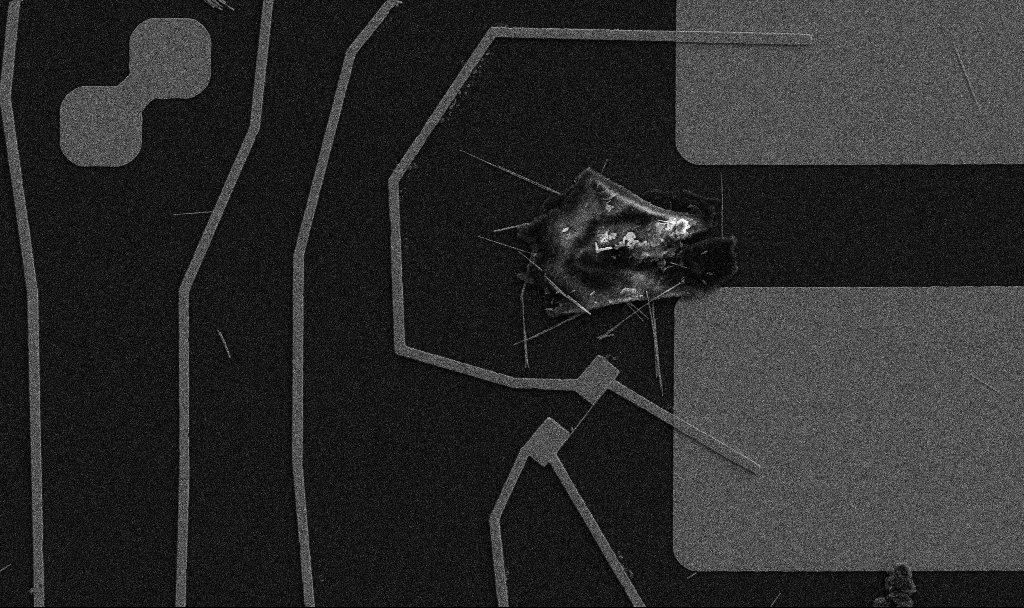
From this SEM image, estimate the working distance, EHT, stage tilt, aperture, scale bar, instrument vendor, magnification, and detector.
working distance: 10.7 mm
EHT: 5 kV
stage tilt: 0°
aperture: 30 µm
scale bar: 10000 nm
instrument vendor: Zeiss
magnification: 5 K X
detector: SE2